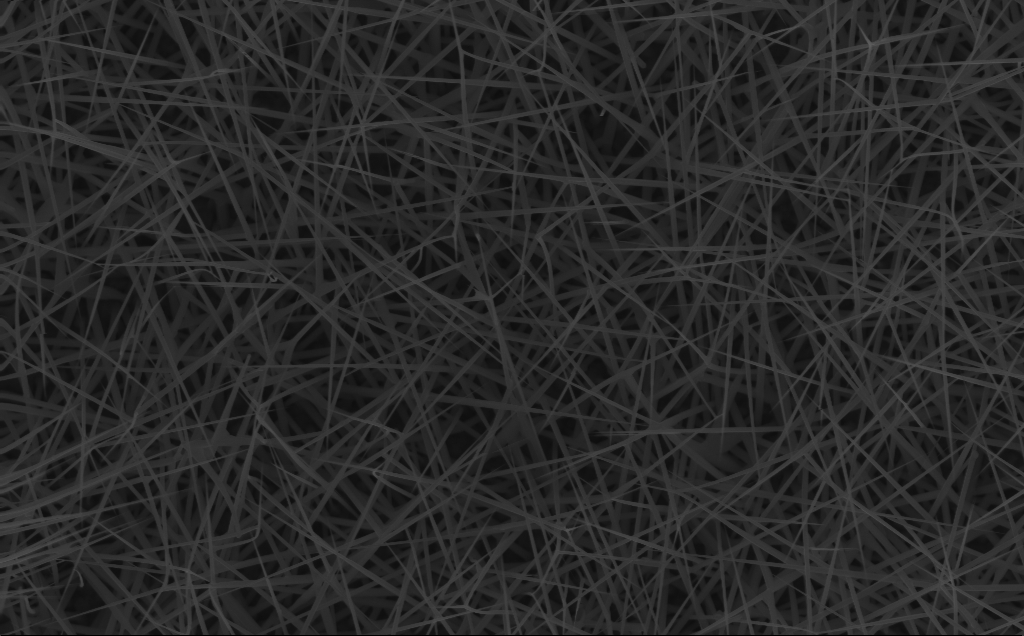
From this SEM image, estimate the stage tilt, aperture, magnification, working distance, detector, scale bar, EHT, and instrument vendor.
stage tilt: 0°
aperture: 30 µm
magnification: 20 K X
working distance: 4 mm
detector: InLens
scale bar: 1000 nm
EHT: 10 kV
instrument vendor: Zeiss